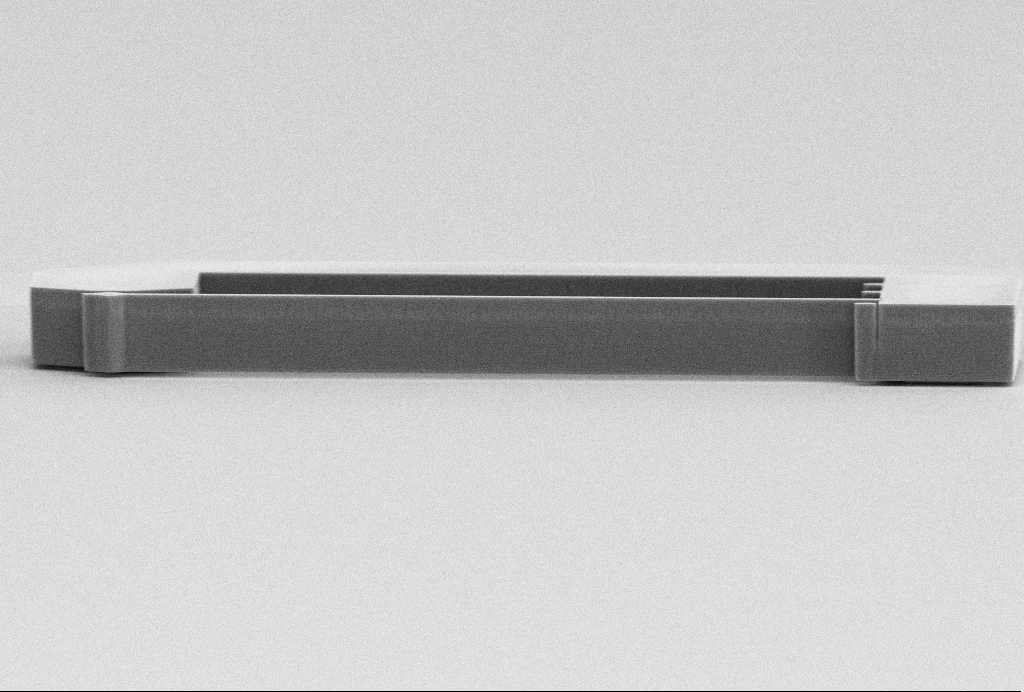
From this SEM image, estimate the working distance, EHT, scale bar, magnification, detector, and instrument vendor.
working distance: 15.7 mm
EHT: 10 kV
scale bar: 20000 nm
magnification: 1.98 K X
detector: InLens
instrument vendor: Zeiss